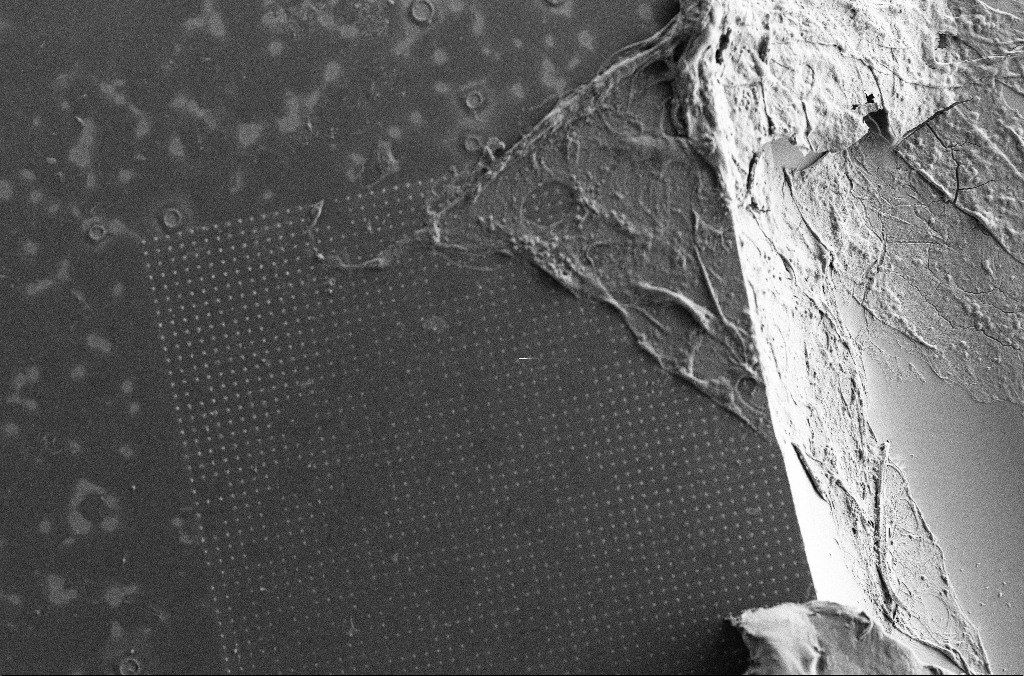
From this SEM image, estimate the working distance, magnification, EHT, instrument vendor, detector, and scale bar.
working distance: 3.7 mm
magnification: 0.15 K X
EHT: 7 kV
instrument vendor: Zeiss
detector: SE2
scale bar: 100000 nm